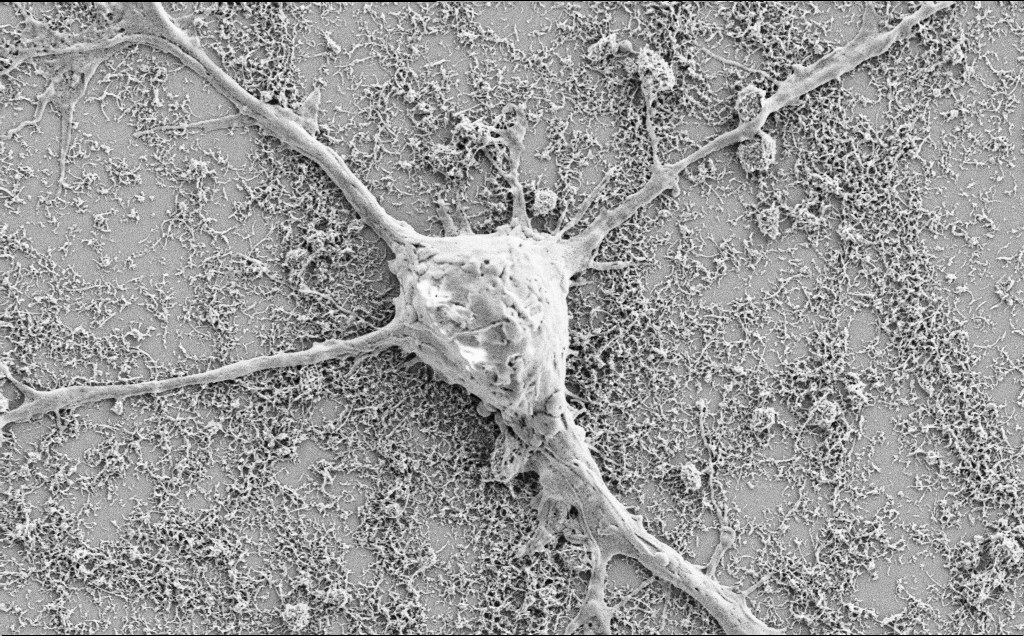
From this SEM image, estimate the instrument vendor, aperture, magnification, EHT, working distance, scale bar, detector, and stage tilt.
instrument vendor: Zeiss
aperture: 30 µm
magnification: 10 K X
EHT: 2 kV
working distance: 7.1 mm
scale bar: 2000 nm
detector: SE2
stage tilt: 0°